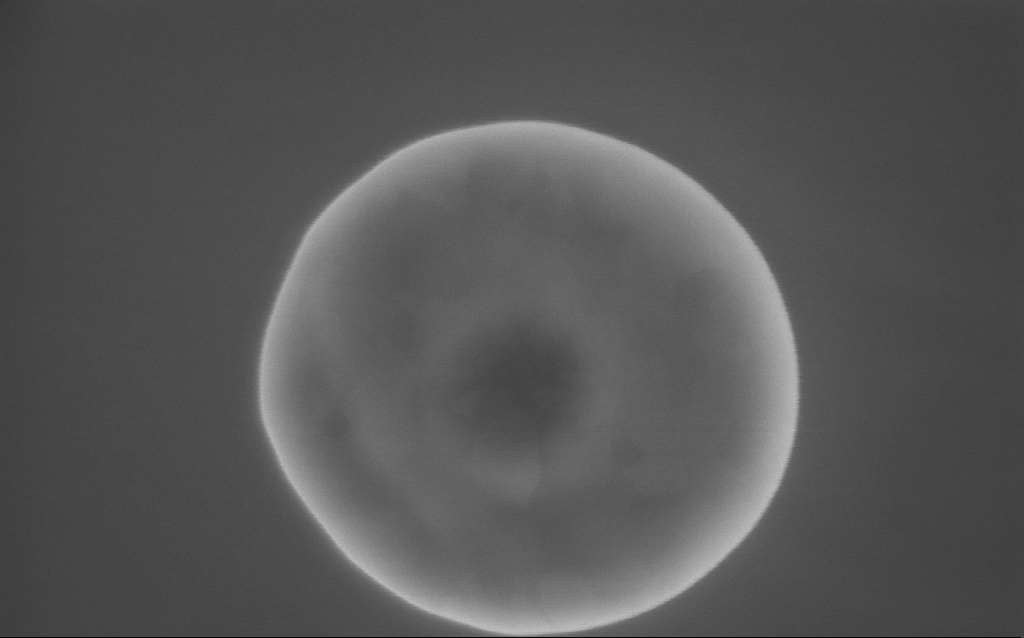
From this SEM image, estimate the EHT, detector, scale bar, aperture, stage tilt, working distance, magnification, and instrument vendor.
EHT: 10 kV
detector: InLens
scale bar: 200 nm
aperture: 30 µm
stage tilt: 0°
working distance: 2 mm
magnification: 157.79 K X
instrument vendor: Zeiss